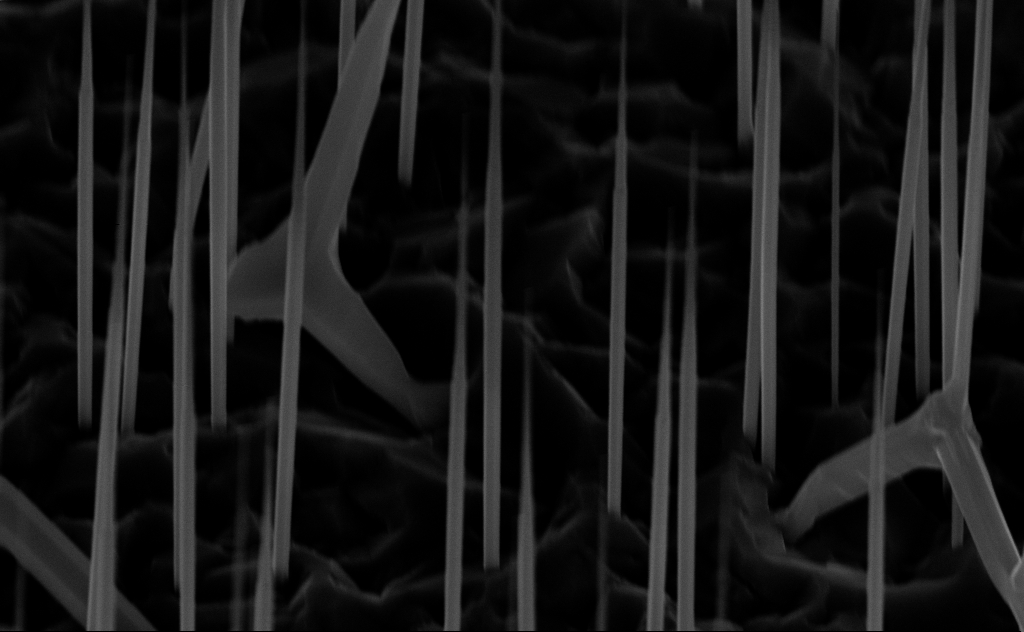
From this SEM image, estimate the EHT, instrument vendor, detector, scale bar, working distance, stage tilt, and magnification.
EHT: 10 kV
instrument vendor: Zeiss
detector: InLens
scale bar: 1000 nm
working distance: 7 mm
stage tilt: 45°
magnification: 40 K X